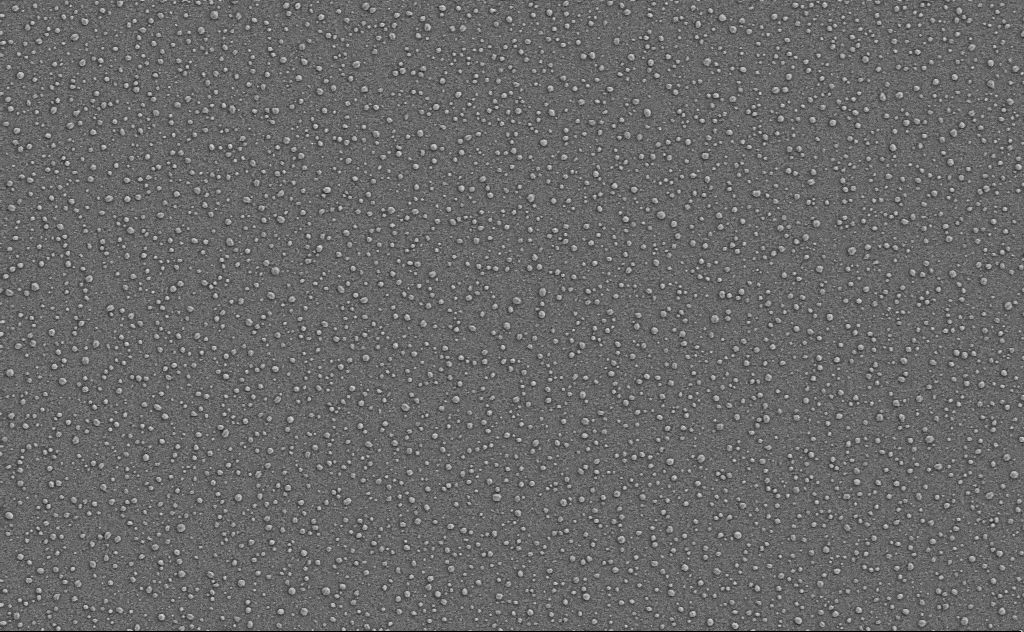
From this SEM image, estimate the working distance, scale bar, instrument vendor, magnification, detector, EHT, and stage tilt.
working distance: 4 mm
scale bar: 2000 nm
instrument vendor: Zeiss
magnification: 20 K X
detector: SE2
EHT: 3 kV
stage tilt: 0°